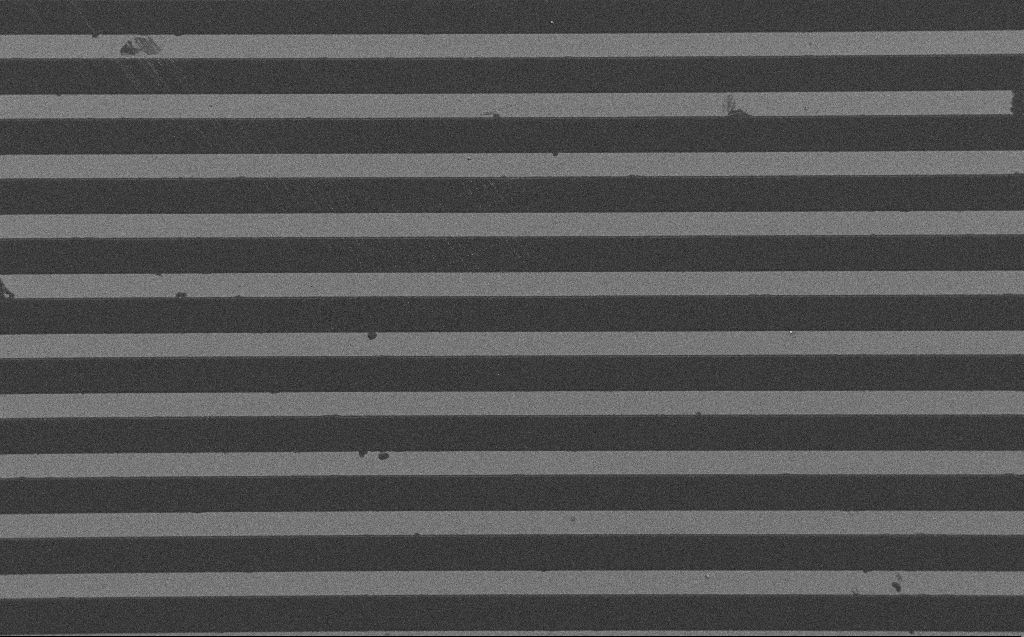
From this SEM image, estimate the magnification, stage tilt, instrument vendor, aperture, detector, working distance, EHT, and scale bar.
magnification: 0.553 K X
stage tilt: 0°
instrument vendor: Zeiss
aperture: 30 µm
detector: SE2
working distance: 4 mm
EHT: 1.2 kV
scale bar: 100000 nm